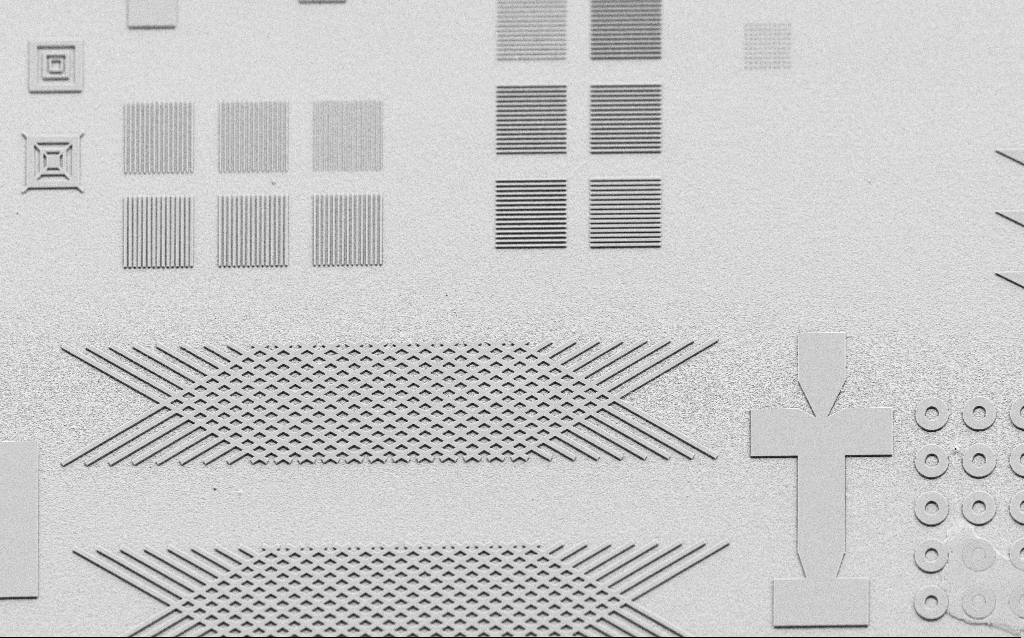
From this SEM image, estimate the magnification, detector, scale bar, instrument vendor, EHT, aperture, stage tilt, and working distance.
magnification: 1.74 K X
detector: SE2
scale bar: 10000 nm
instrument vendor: Zeiss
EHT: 3 kV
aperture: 30 µm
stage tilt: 45°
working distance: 8 mm